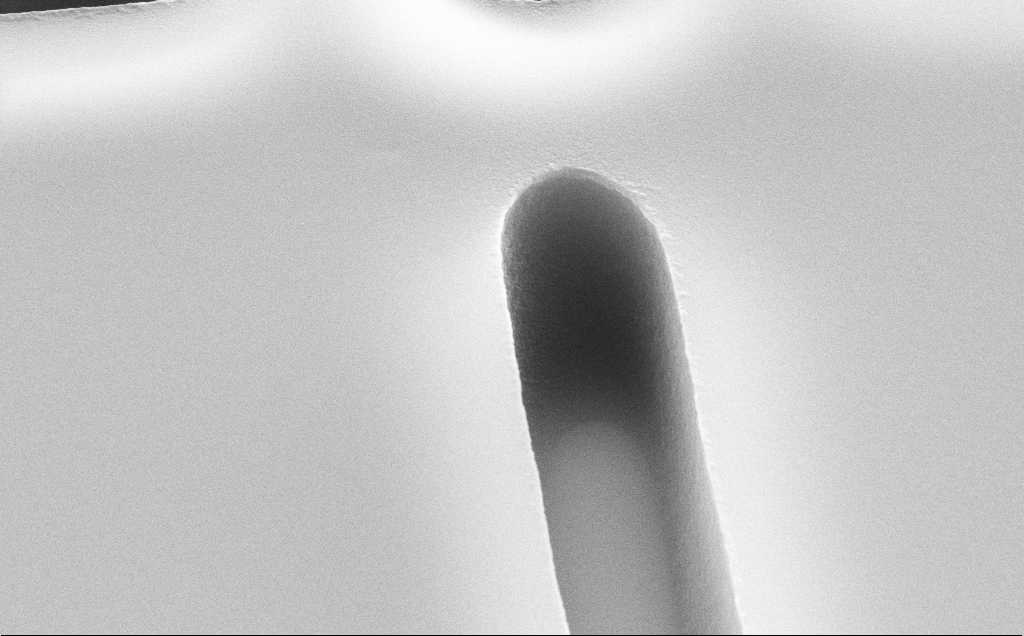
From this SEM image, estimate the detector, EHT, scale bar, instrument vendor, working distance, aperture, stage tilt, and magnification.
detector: SE2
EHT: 10 kV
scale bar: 1000 nm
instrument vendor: Zeiss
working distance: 11 mm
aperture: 30 µm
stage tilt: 45°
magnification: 38.82 K X